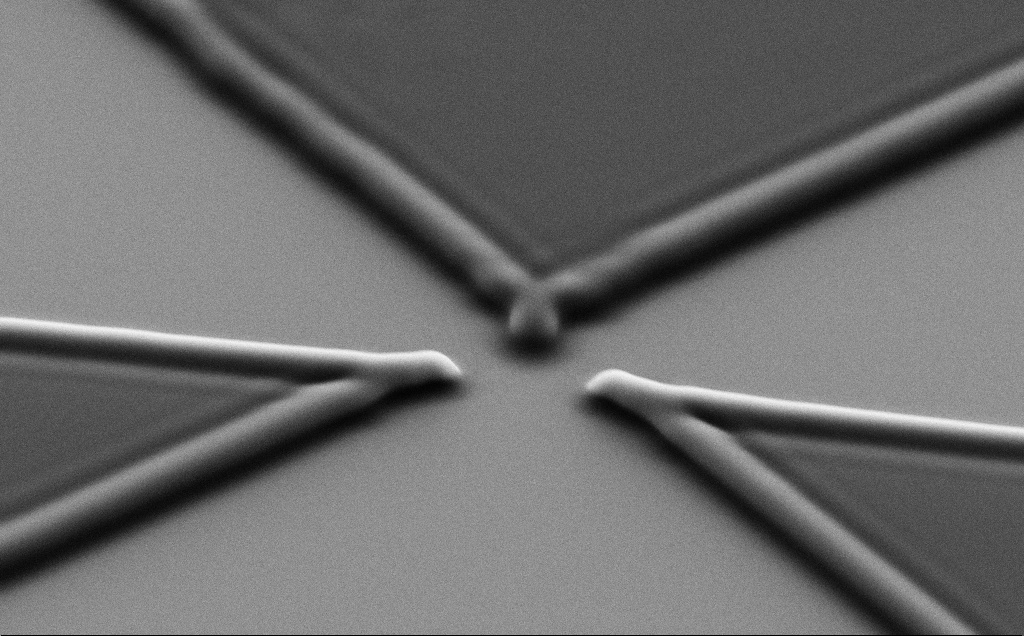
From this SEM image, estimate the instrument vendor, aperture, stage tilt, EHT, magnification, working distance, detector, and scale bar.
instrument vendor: Zeiss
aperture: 30 µm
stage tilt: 35°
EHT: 7 kV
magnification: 8.77 K X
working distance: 5 mm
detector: SE2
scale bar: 2000 nm